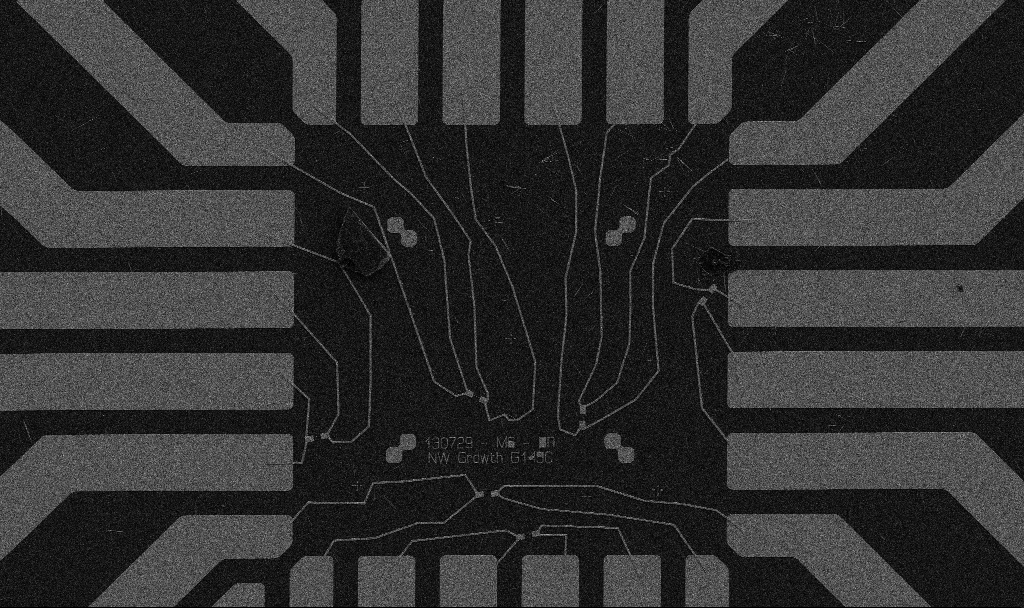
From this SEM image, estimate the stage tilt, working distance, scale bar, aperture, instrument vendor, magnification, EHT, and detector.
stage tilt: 0°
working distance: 10.7 mm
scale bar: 20000 nm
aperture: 30 µm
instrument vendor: Zeiss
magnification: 1 K X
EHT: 5 kV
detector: SE2